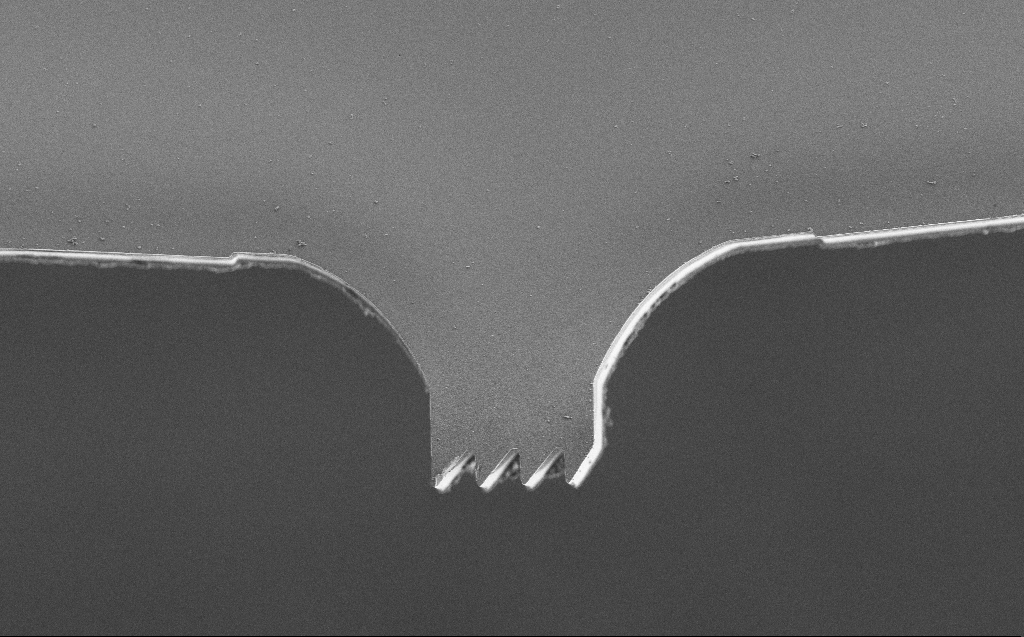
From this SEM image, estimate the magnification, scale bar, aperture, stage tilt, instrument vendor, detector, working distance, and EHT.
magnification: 1.46 K X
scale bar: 10000 nm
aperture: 30 µm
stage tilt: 0°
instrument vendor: Zeiss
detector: SE2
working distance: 4 mm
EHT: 3 kV